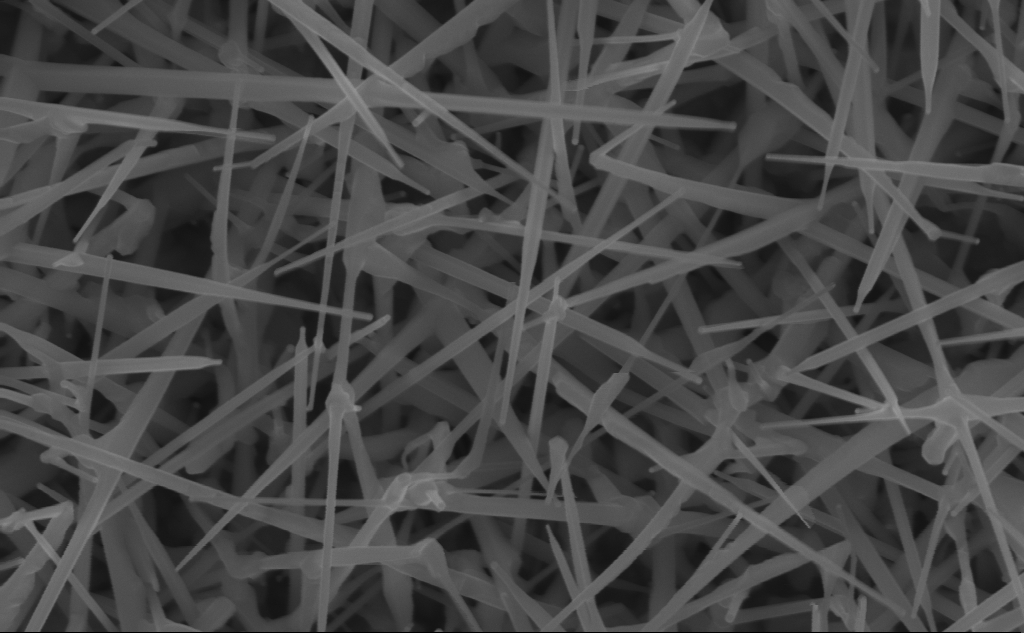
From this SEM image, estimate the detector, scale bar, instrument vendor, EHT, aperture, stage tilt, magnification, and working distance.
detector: InLens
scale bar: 200 nm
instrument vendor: Zeiss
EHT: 10 kV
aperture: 30 µm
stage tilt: -0°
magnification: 78.16 K X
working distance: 7 mm